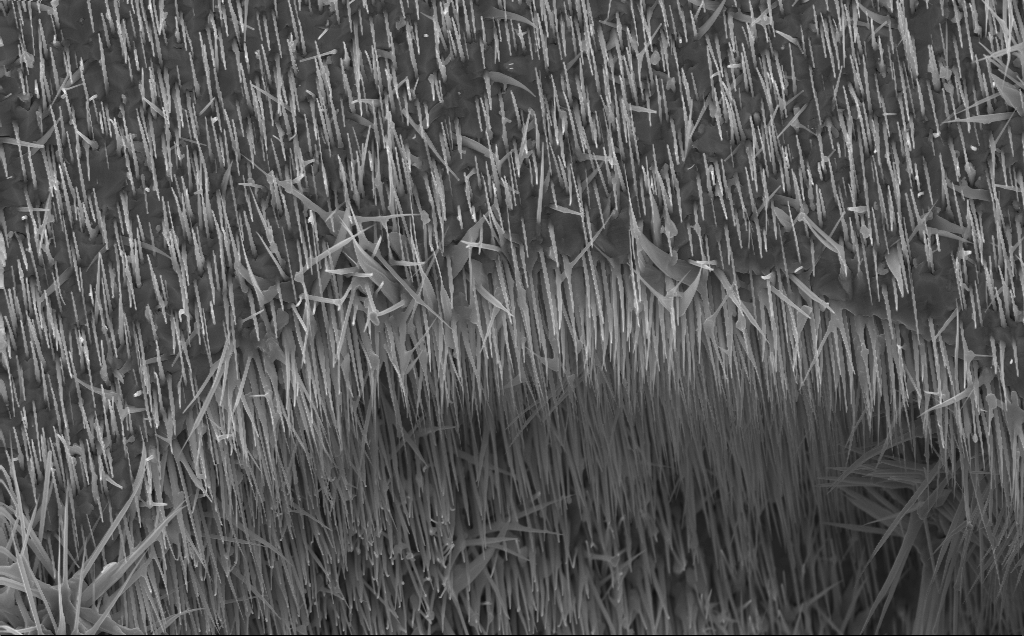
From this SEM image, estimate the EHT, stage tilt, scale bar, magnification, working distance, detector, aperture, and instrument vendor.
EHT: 10 kV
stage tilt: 0°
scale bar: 2000 nm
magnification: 12.06 K X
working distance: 7 mm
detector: InLens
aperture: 30 µm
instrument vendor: Zeiss